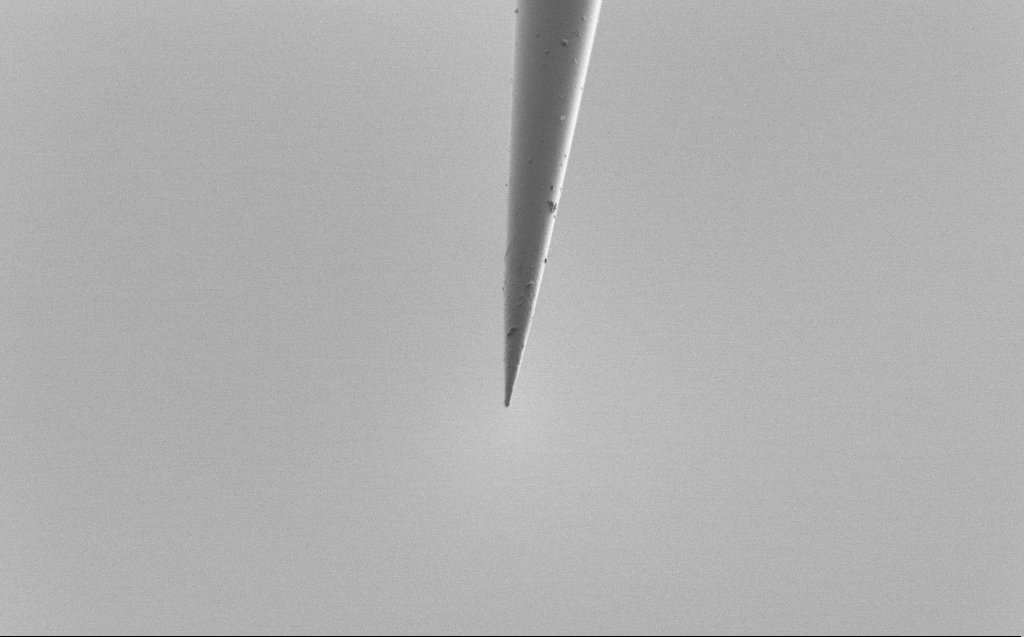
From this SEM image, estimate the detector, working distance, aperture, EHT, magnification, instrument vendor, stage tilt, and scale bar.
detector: SE2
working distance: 5 mm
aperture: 30 µm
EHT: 1 kV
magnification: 10 K X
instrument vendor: Zeiss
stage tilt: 45°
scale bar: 2000 nm